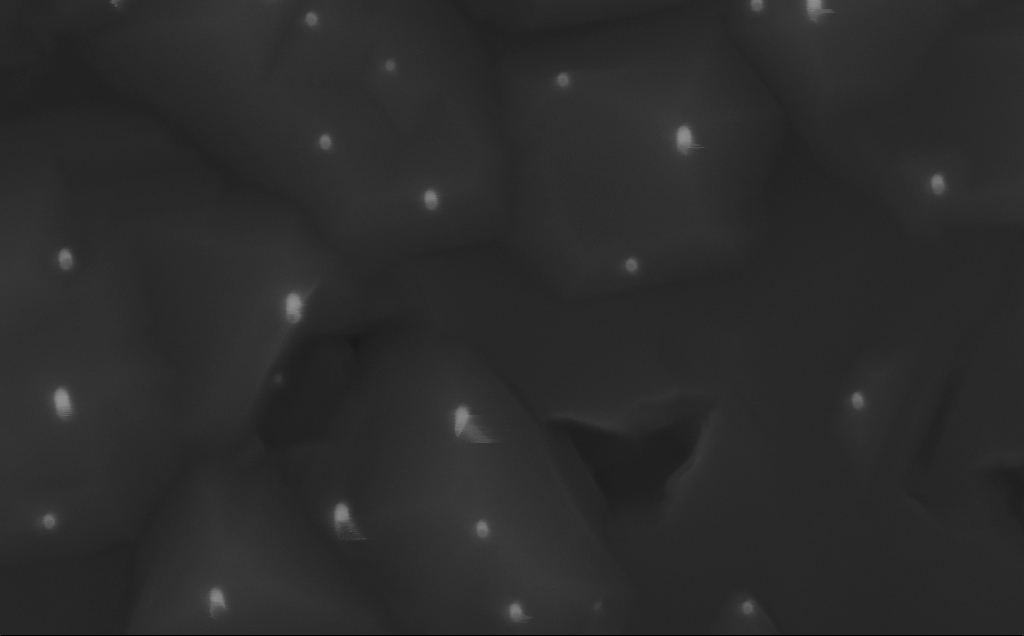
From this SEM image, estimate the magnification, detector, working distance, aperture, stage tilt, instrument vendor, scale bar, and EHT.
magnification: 150 K X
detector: InLens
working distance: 3 mm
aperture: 30 µm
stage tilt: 0°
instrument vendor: Zeiss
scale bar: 100 nm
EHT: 10 kV